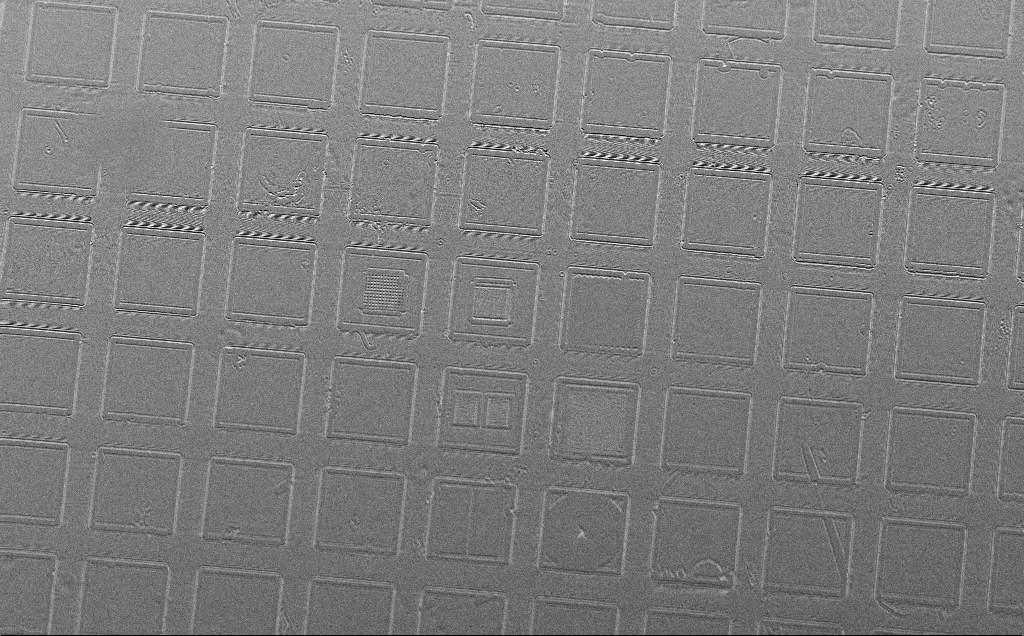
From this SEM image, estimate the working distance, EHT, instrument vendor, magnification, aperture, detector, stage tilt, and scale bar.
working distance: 10 mm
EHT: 5 kV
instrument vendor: Zeiss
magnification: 0.292 K X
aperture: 30 µm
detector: SE2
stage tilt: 45°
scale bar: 100000 nm